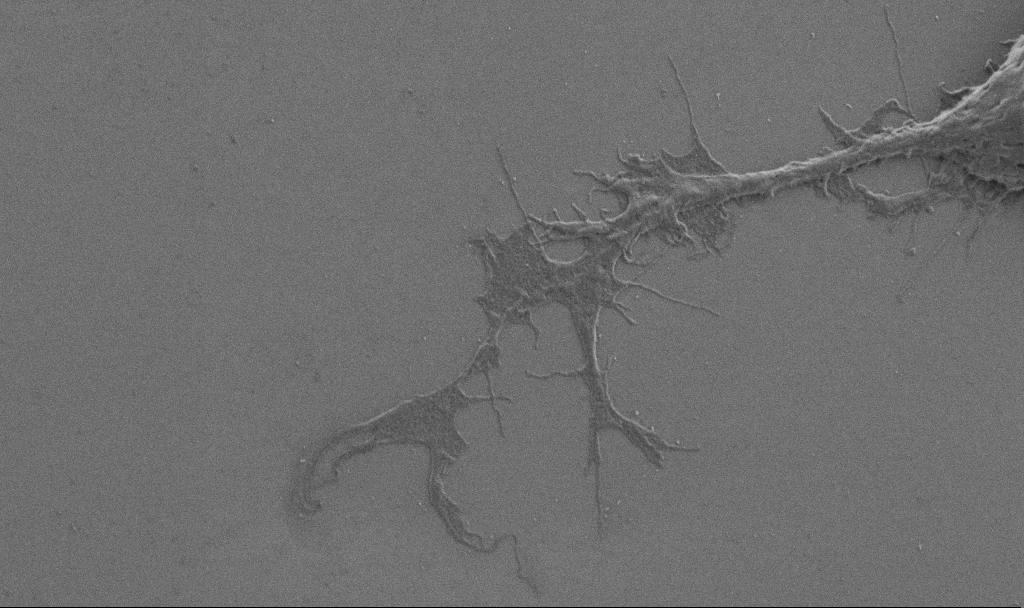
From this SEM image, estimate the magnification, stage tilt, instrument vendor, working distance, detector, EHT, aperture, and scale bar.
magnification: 10 K X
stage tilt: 0°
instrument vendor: Zeiss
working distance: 6.9 mm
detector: SE2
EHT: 1 kV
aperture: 30 µm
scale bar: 2000 nm